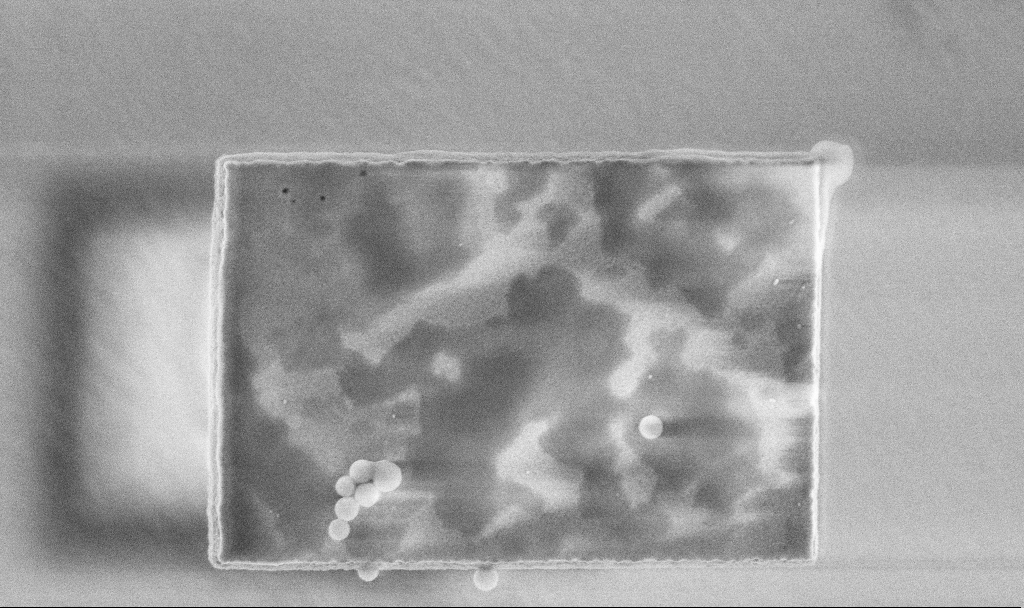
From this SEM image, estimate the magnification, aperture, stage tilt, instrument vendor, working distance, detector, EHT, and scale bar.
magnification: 50 K X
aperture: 30 µm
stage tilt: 0°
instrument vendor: Zeiss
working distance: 3.3 mm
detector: InLens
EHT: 3 kV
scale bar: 1000 nm